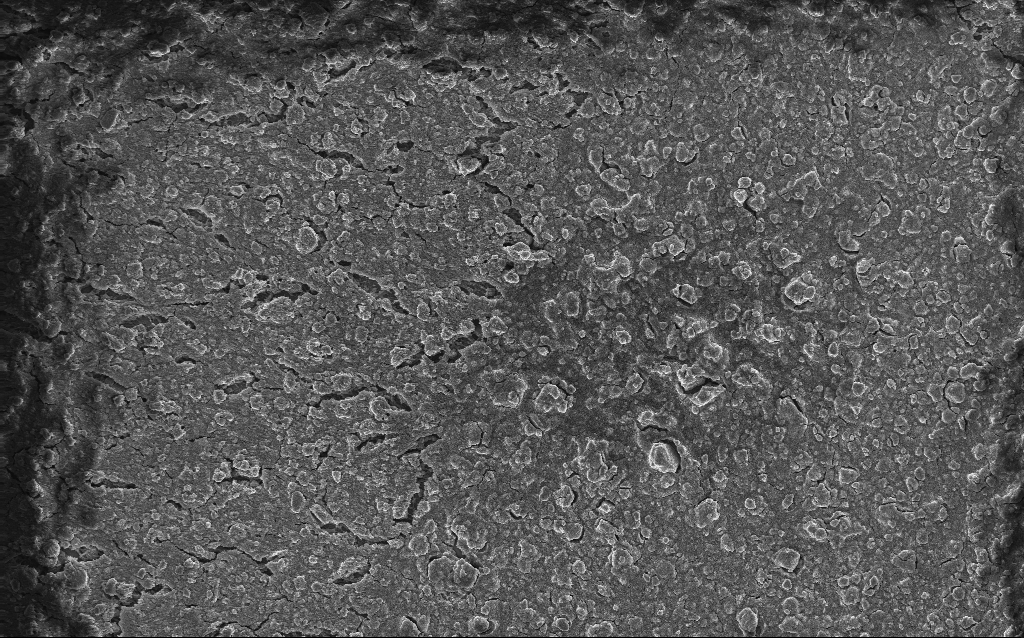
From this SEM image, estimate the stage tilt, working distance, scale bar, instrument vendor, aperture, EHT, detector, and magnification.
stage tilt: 0°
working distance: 2.6 mm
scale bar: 20000 nm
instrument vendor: Zeiss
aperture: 30 µm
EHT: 10 kV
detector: InLens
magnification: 0.792 K X